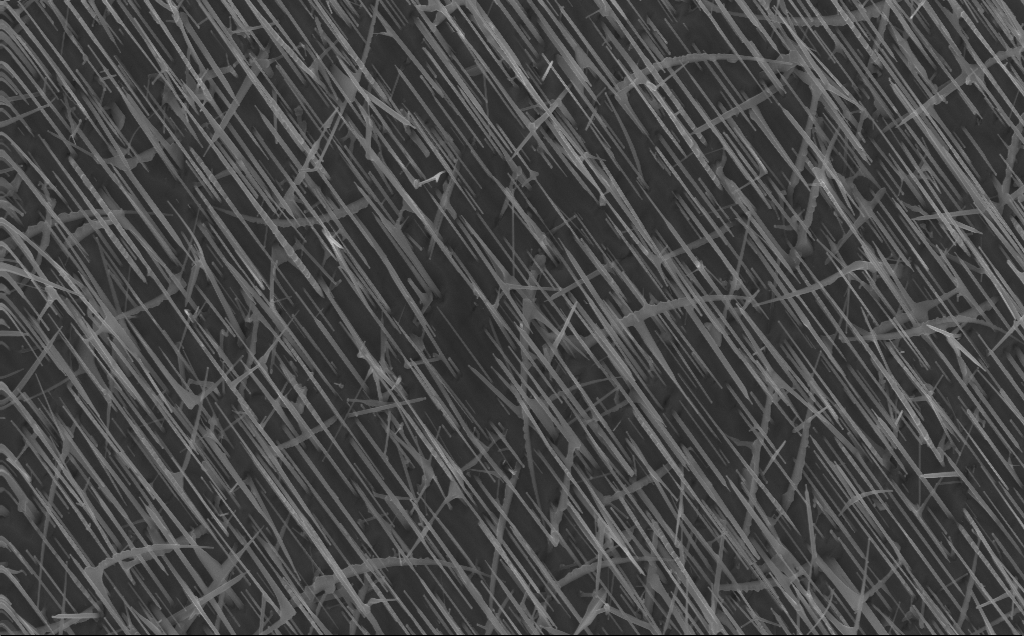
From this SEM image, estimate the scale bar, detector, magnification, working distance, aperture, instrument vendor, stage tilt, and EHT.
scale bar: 2000 nm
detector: InLens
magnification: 20 K X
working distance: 4 mm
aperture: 30 µm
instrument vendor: Zeiss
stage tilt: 0°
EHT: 10 kV